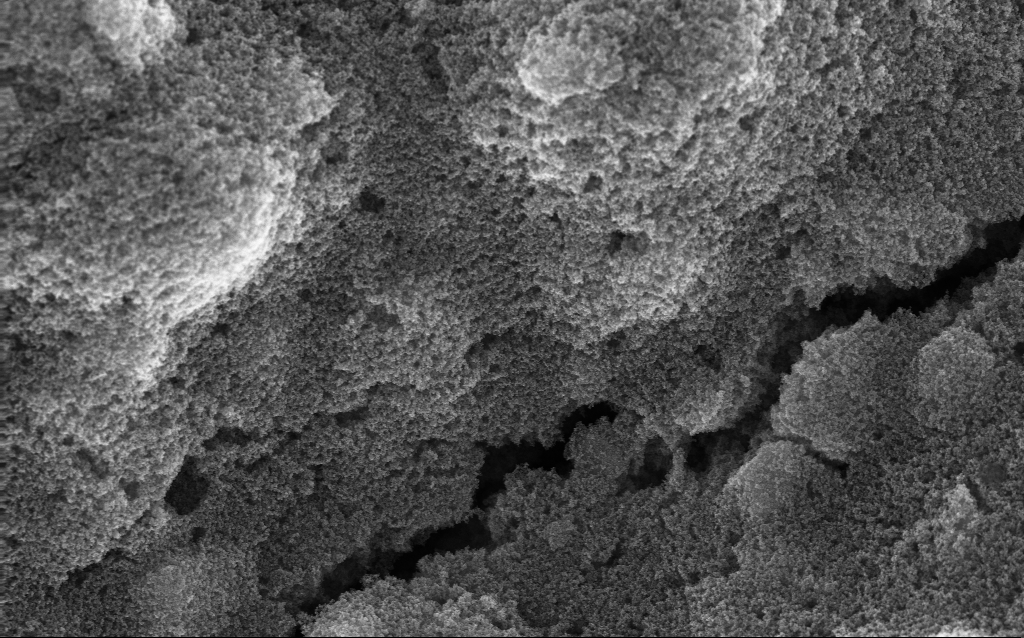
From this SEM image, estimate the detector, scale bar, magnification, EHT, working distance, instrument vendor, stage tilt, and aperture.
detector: InLens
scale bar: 1000 nm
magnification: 23.9 K X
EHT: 10 kV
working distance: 2.7 mm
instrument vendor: Zeiss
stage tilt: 0°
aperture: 30 µm